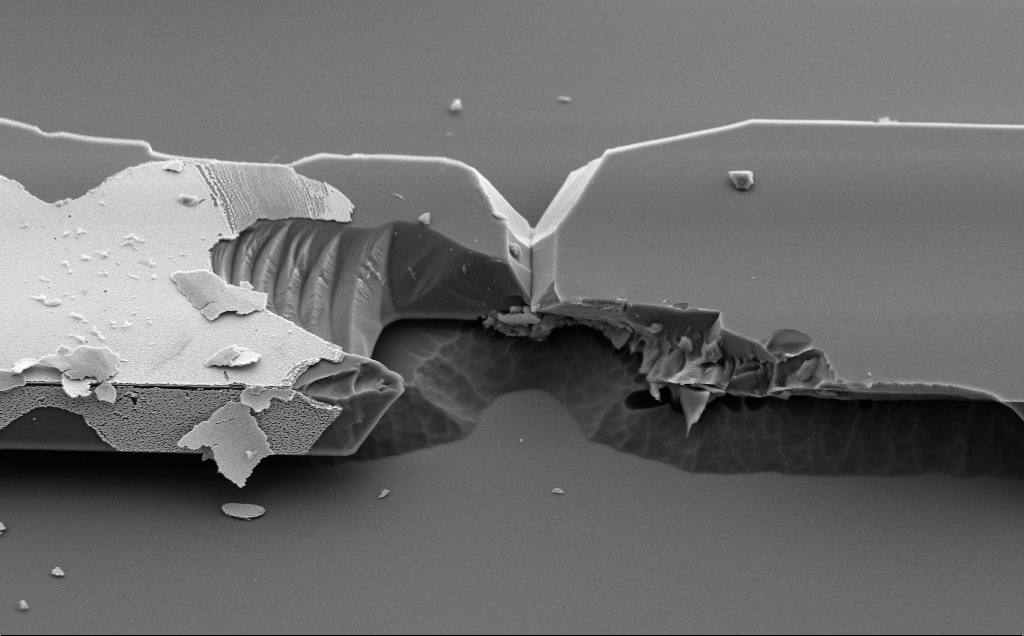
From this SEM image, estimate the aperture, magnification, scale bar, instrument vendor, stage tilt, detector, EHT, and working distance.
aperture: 30 µm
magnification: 8.69 K X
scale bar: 2000 nm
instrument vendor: Zeiss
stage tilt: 50°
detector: SE2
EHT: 5 kV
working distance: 10 mm